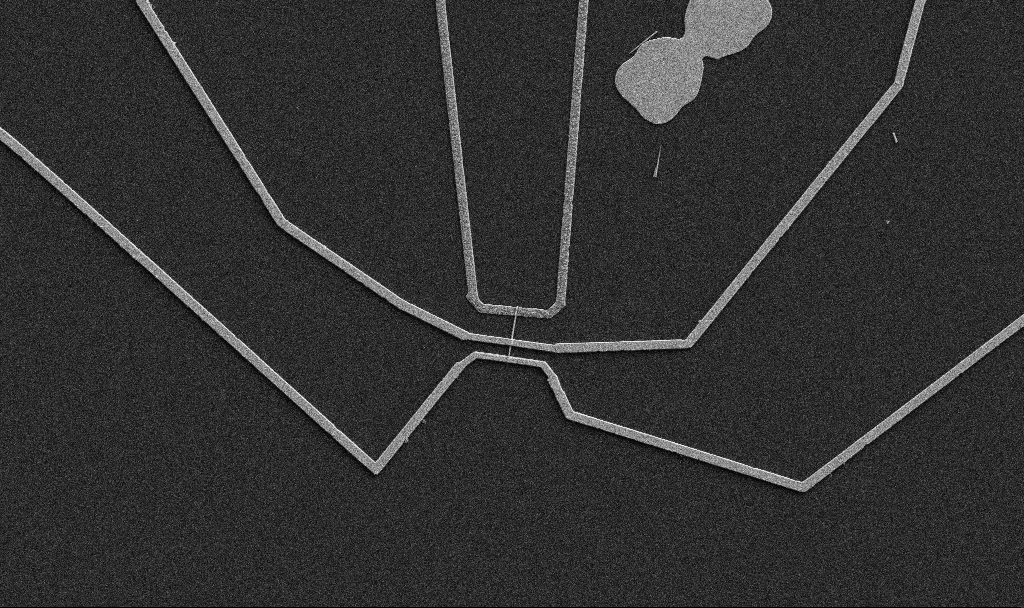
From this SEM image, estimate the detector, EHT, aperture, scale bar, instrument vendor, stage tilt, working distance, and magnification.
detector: SE2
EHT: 5 kV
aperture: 30 µm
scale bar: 10000 nm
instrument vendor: Zeiss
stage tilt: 0°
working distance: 10.7 mm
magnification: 5 K X